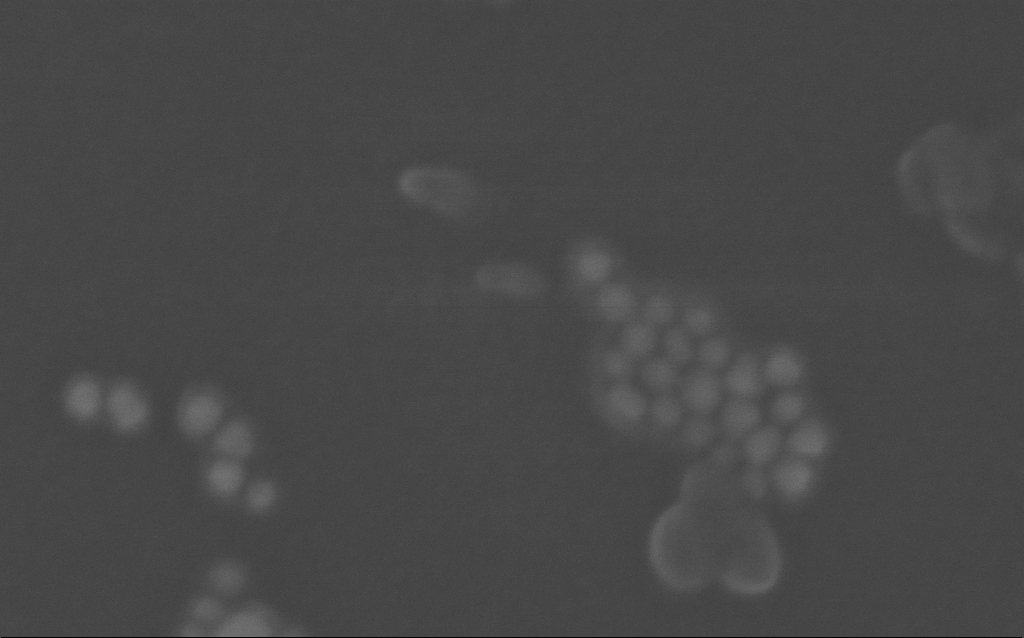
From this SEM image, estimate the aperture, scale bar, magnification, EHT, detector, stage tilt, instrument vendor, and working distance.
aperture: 30 µm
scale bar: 20 nm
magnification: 935.13 K X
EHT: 10 kV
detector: InLens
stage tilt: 0°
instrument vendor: Zeiss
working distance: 2.9 mm